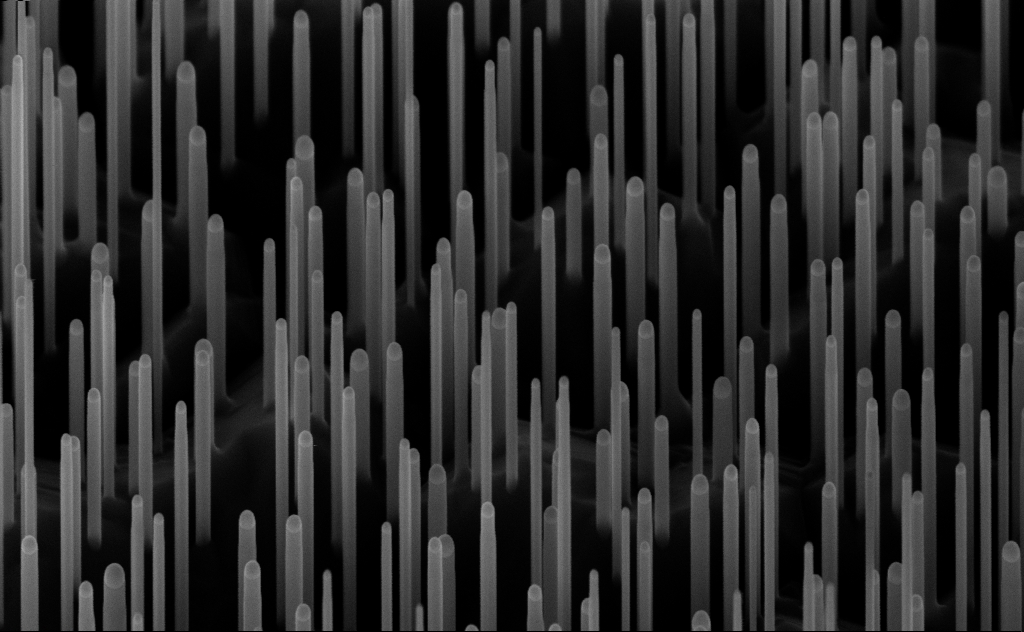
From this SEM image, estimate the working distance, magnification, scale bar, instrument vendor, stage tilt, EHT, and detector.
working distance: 7 mm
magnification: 80 K X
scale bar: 200 nm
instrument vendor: Zeiss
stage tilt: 45°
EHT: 10 kV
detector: InLens